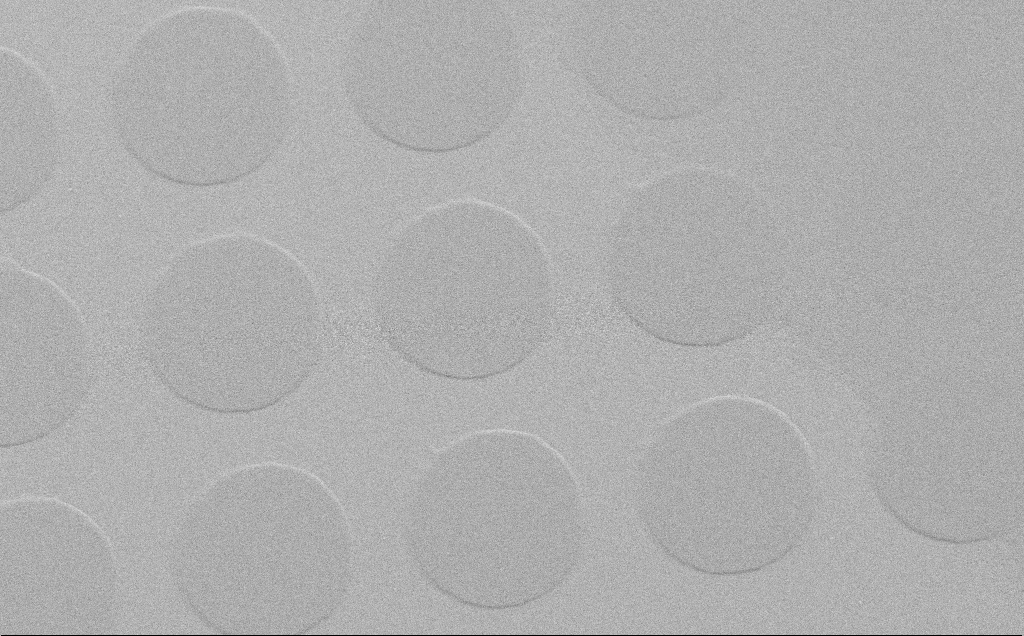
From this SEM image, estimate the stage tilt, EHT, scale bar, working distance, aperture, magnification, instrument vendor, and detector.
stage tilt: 40.4°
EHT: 10 kV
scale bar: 10000 nm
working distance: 6 mm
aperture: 30 µm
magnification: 1.63 K X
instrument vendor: Zeiss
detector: SE2